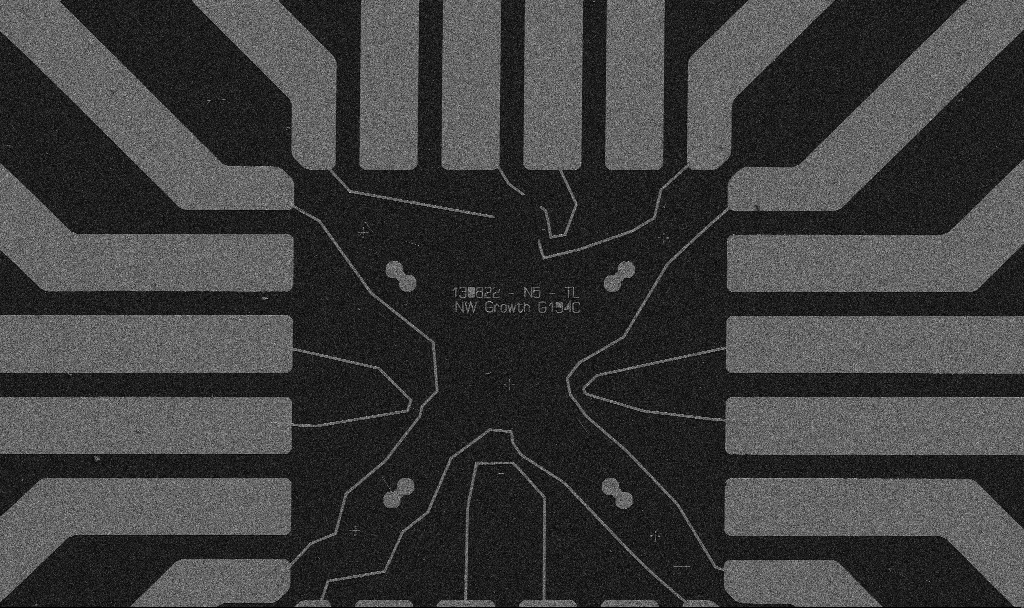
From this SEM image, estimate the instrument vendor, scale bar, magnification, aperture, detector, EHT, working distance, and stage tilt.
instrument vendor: Zeiss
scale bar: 20000 nm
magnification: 1 K X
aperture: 30 µm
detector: SE2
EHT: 5 kV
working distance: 10.7 mm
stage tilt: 0°